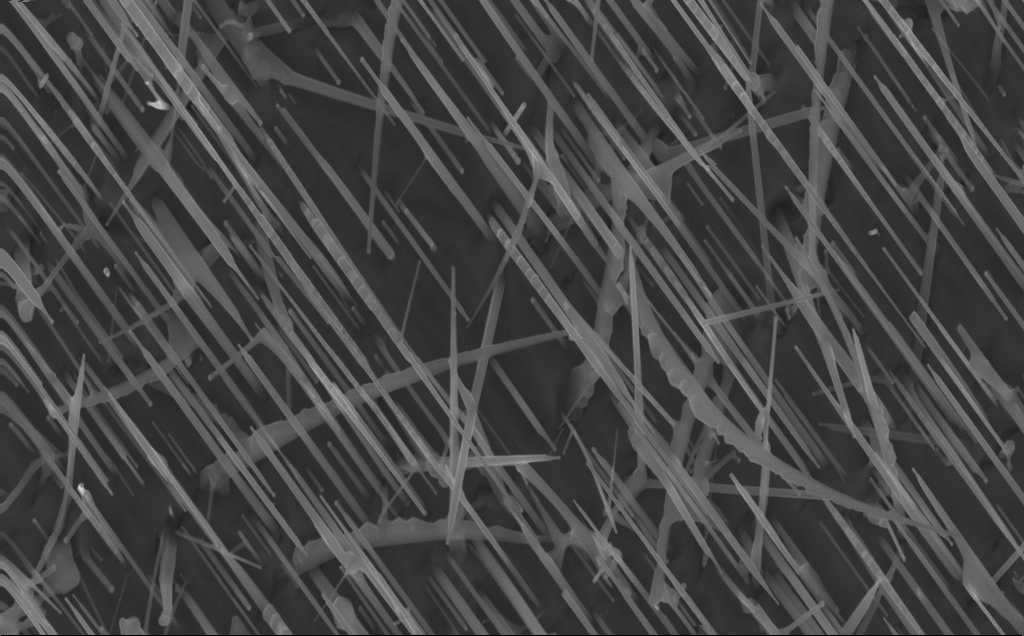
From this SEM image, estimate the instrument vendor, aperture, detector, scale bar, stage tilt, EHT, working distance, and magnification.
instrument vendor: Zeiss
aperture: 30 µm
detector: InLens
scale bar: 1000 nm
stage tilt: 0°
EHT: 10 kV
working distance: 4 mm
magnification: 40 K X